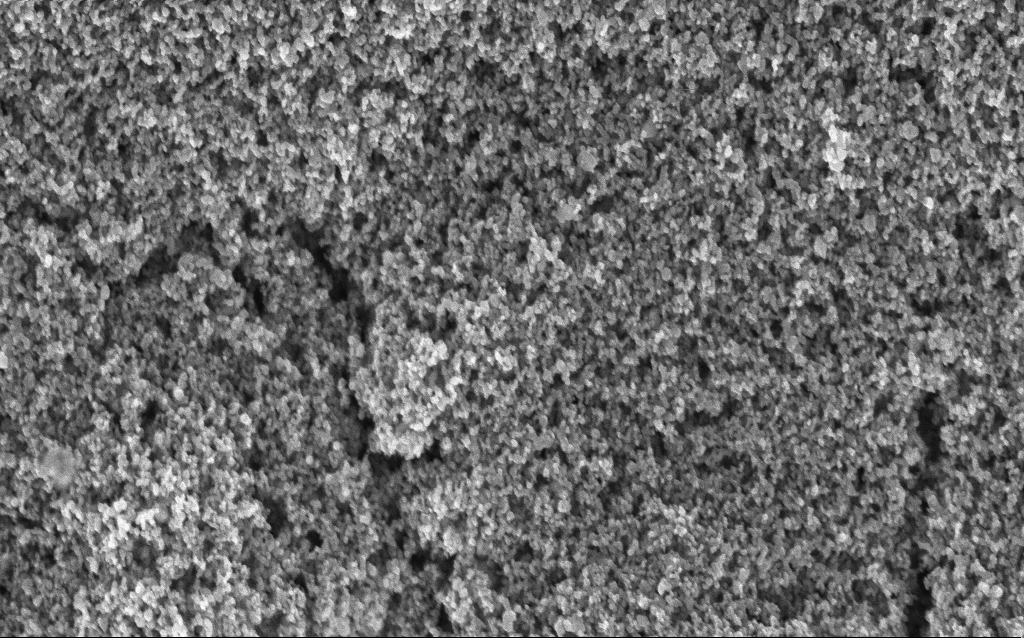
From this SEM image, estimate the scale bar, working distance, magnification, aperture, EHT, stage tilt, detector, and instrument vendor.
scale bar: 1000 nm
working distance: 2.7 mm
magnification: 65.04 K X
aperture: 30 µm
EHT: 5 kV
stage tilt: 0°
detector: InLens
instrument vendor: Zeiss